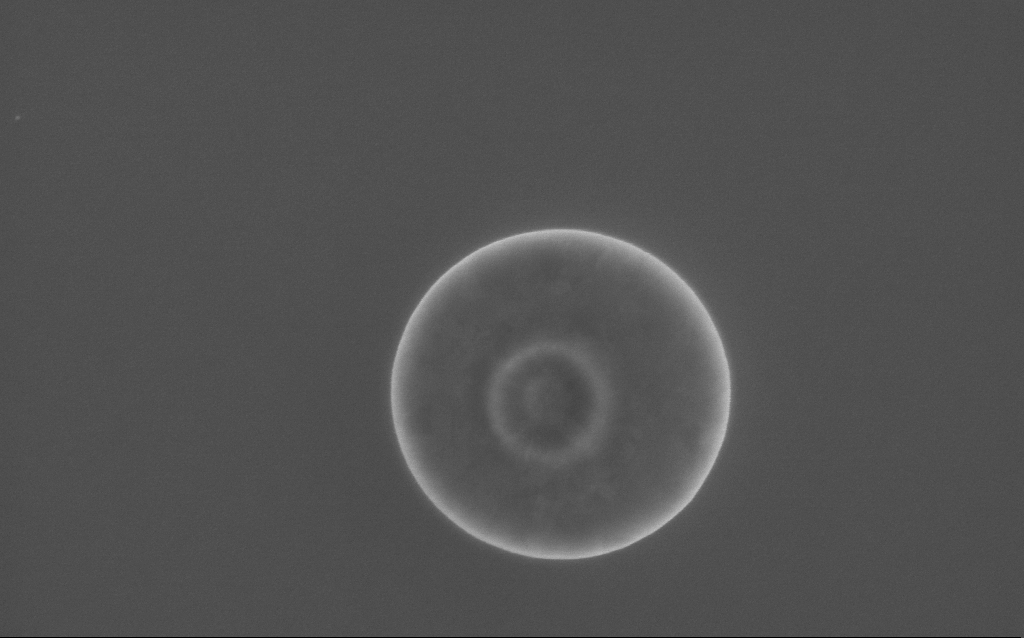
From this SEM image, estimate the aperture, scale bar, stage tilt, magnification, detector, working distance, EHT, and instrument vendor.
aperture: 30 µm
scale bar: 200 nm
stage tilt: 0°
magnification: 90.55 K X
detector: InLens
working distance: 2 mm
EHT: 5 kV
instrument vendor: Zeiss